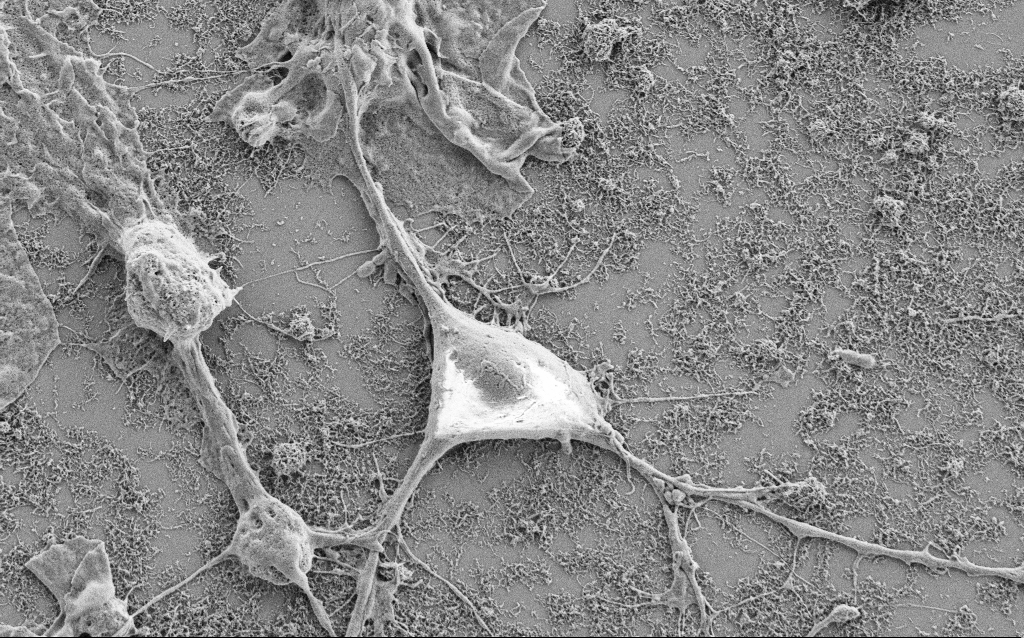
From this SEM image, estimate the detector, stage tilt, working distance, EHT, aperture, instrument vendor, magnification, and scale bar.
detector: SE2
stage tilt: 0°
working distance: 6.8 mm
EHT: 2 kV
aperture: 30 µm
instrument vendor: Zeiss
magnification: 7.5 K X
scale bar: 2000 nm